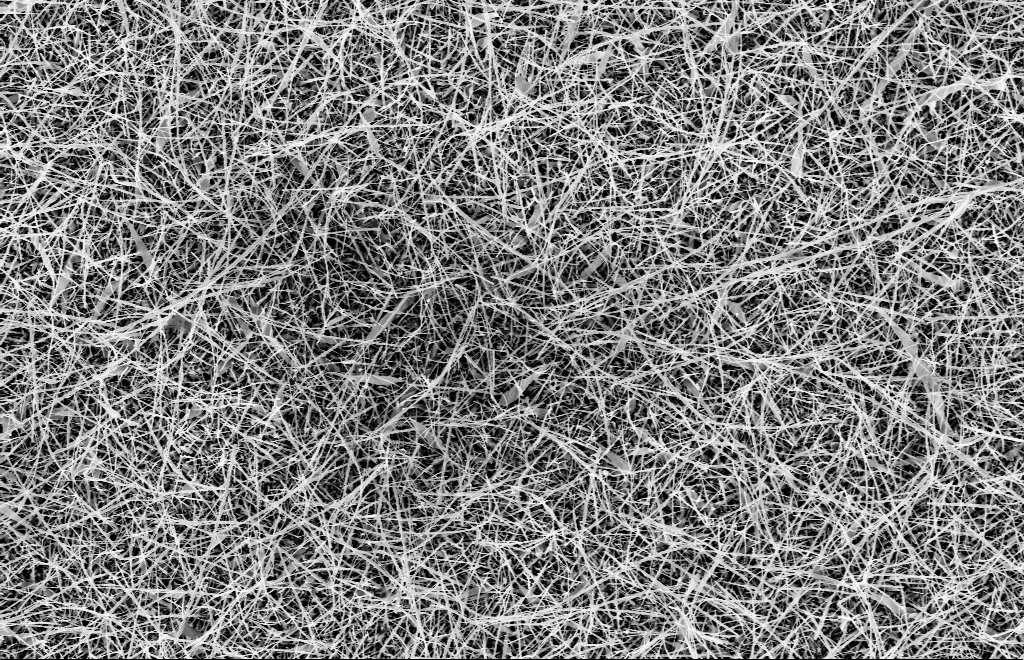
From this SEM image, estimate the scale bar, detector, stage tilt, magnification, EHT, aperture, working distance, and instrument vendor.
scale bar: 2000 nm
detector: InLens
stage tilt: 0°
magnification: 5 K X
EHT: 10 kV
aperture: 30 µm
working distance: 14 mm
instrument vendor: Zeiss